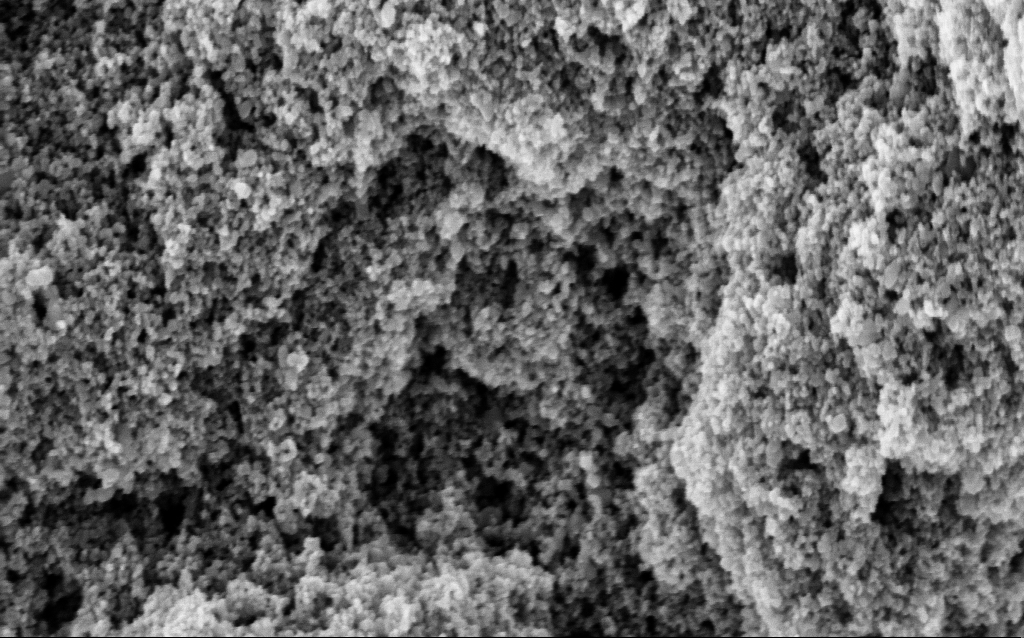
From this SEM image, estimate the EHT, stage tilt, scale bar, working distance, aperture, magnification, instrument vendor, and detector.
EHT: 5 kV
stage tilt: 0°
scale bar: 200 nm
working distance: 9.6 mm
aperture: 30 µm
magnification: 76.33 K X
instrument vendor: Zeiss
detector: InLens